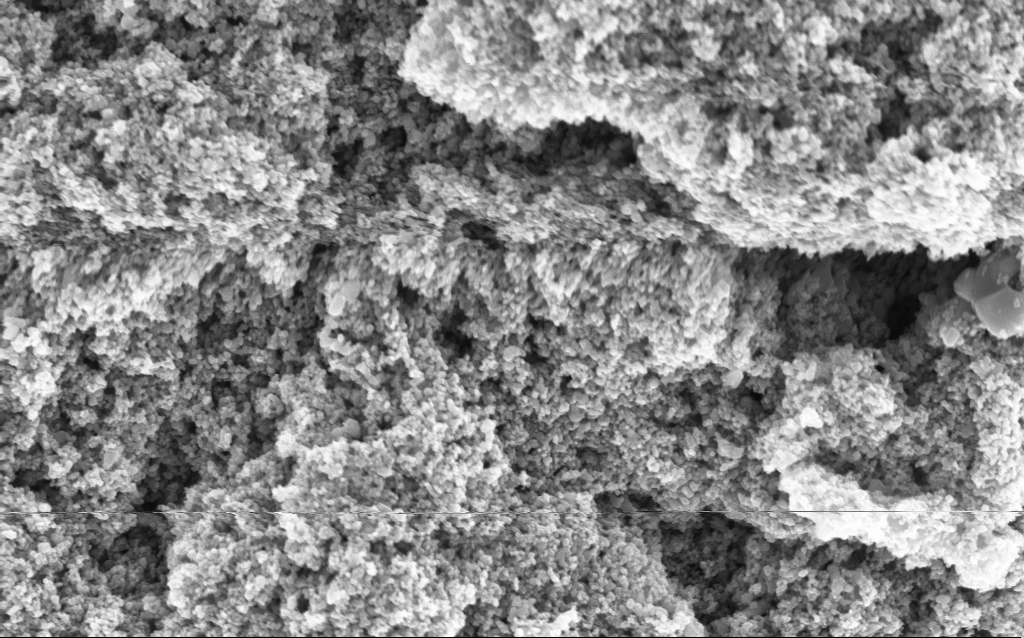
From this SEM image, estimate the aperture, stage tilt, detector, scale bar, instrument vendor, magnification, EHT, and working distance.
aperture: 30 µm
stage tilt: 0°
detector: InLens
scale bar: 1000 nm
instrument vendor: Zeiss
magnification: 68.65 K X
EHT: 5 kV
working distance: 4.7 mm